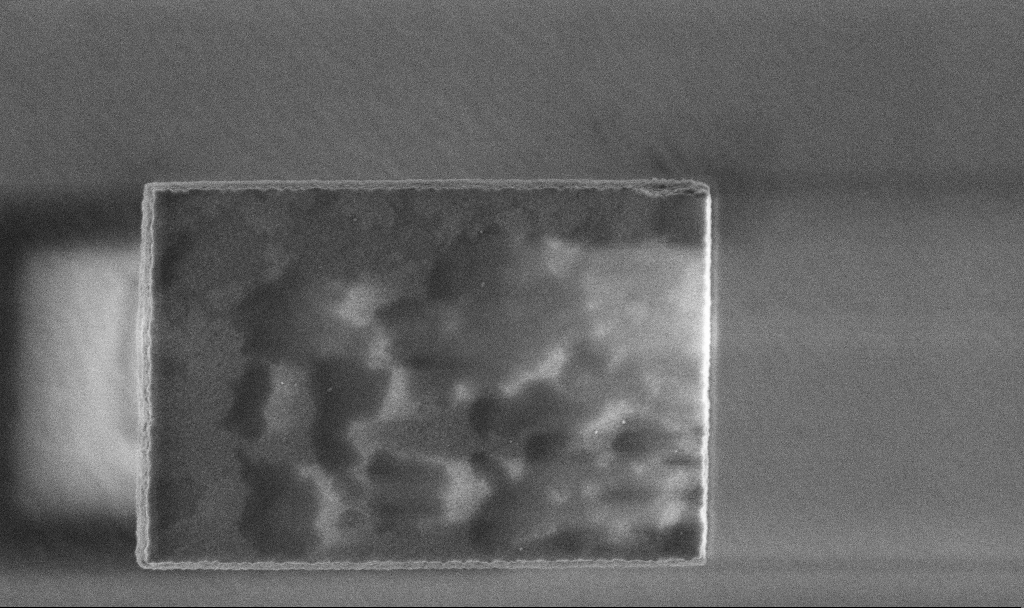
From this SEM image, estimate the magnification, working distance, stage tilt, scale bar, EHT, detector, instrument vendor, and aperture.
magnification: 46.81 K X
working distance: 3.3 mm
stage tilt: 0°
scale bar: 1000 nm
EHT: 3 kV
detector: InLens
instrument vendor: Zeiss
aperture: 30 µm